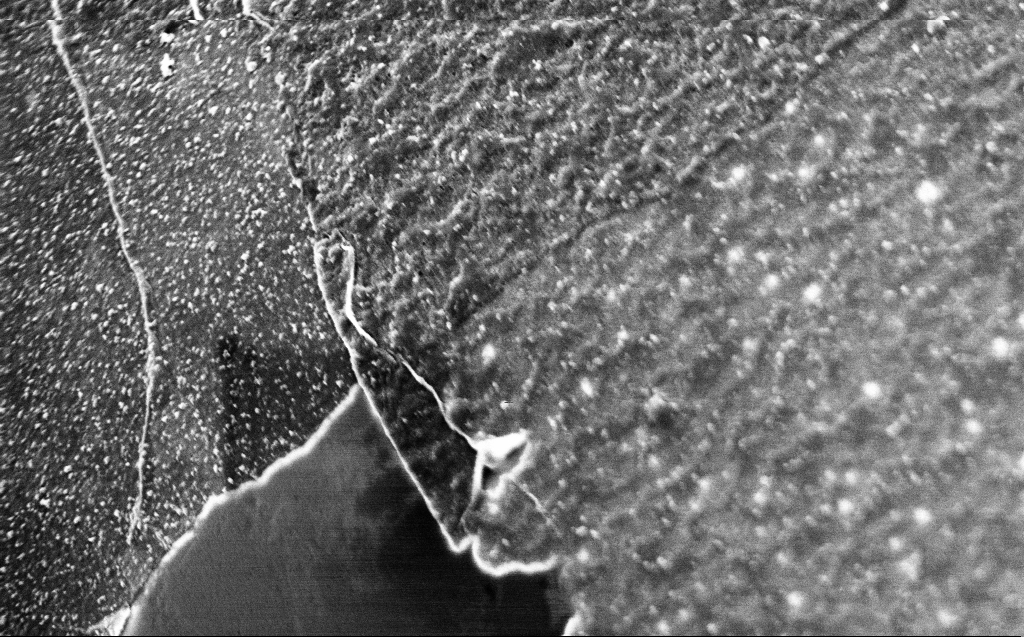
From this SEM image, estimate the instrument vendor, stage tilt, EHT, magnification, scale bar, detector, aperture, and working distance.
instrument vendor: Zeiss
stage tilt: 45°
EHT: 2 kV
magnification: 50 K X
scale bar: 1000 nm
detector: InLens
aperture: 30 µm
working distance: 3 mm